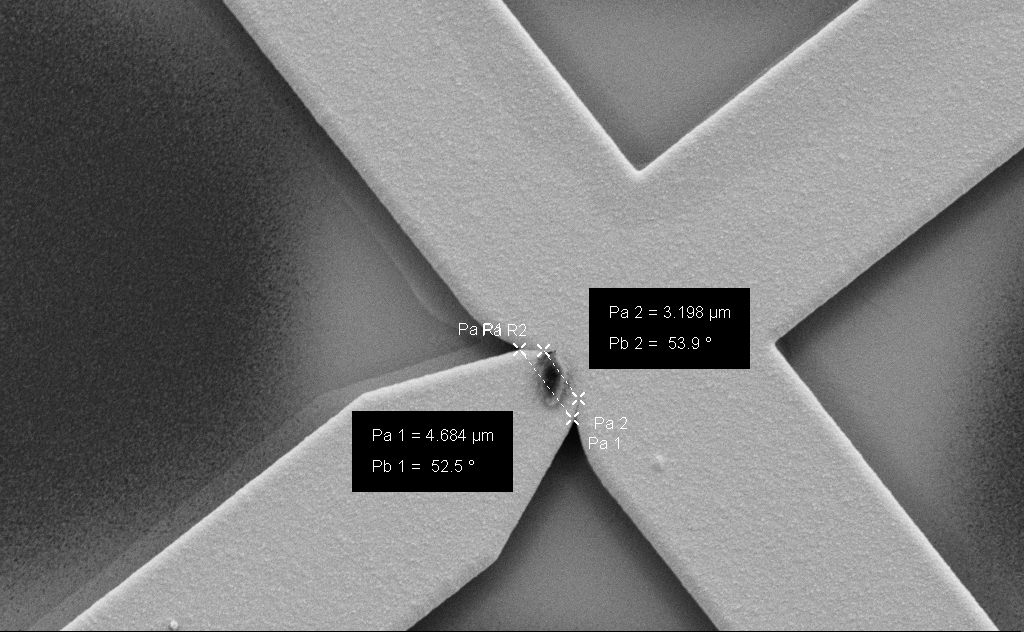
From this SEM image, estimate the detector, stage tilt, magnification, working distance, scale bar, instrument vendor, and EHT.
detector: SE2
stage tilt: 0°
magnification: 6.82 K X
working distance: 9 mm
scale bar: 10000 nm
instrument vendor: Zeiss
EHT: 5 kV